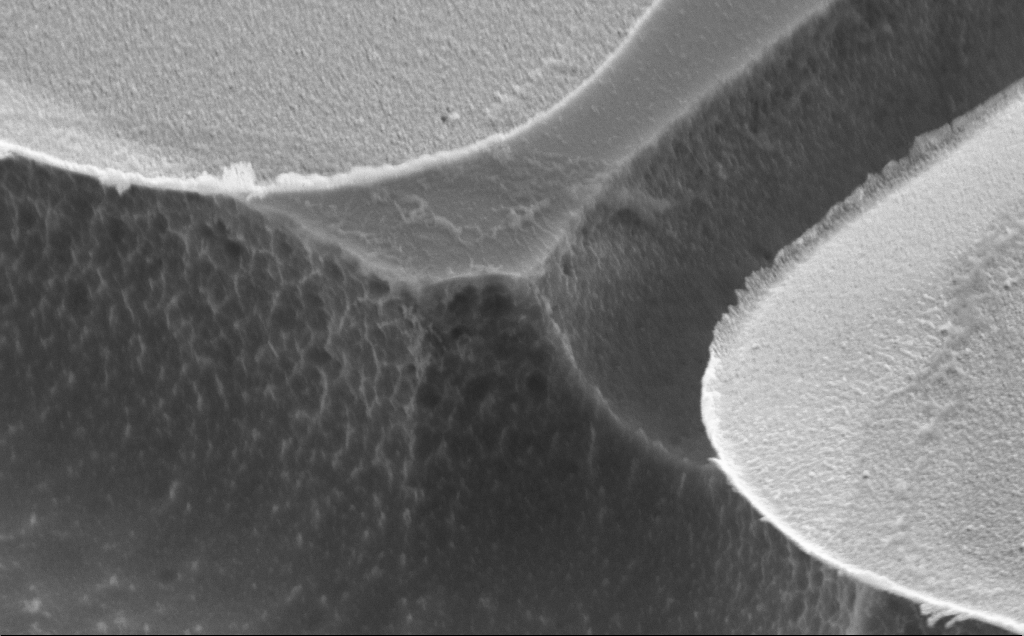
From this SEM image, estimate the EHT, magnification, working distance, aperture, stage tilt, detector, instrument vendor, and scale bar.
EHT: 5 kV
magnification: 49.49 K X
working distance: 10 mm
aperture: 30 µm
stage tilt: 50°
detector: SE2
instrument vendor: Zeiss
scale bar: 1000 nm